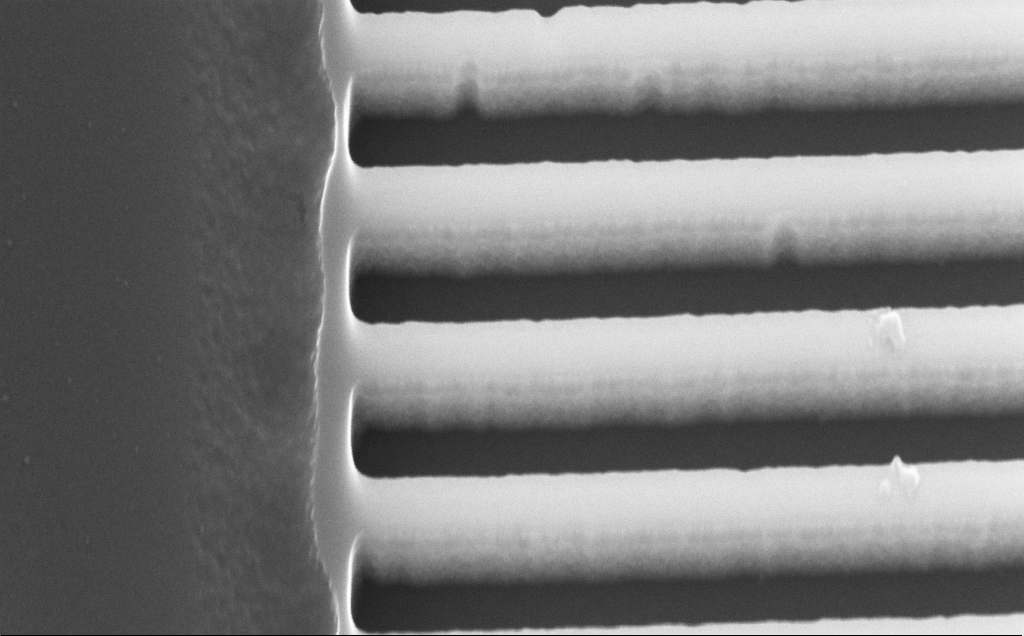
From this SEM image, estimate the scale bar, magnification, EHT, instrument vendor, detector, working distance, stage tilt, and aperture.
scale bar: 200 nm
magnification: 112.75 K X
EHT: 10 kV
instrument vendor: Zeiss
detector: InLens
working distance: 4 mm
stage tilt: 30°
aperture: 30 µm